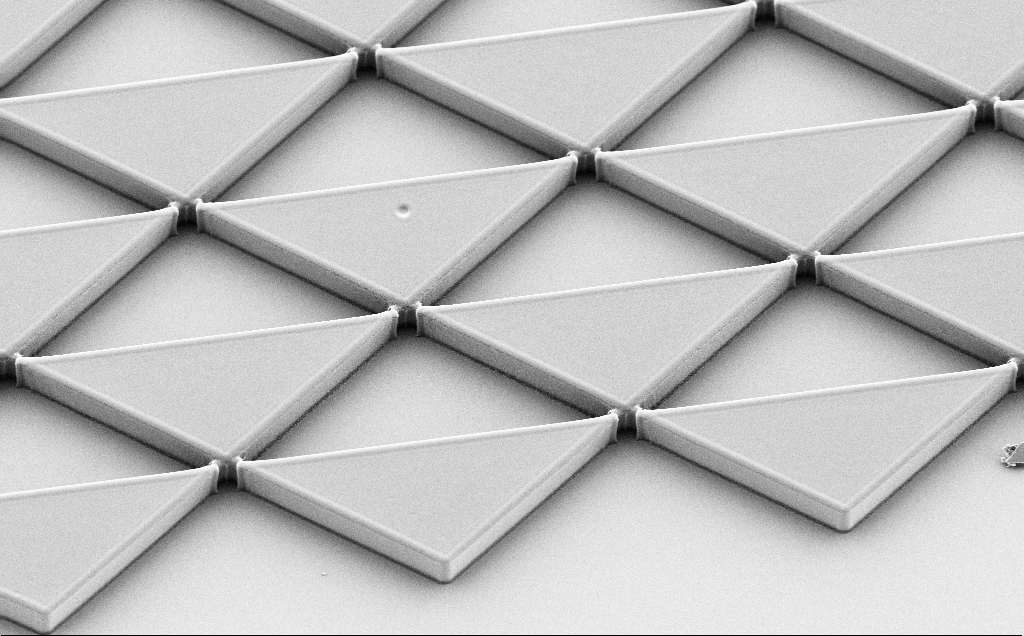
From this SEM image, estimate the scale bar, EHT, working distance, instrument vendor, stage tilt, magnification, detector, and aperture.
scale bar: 10000 nm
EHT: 5 kV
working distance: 8 mm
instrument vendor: Zeiss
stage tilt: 30°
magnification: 1.58 K X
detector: SE2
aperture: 30 µm